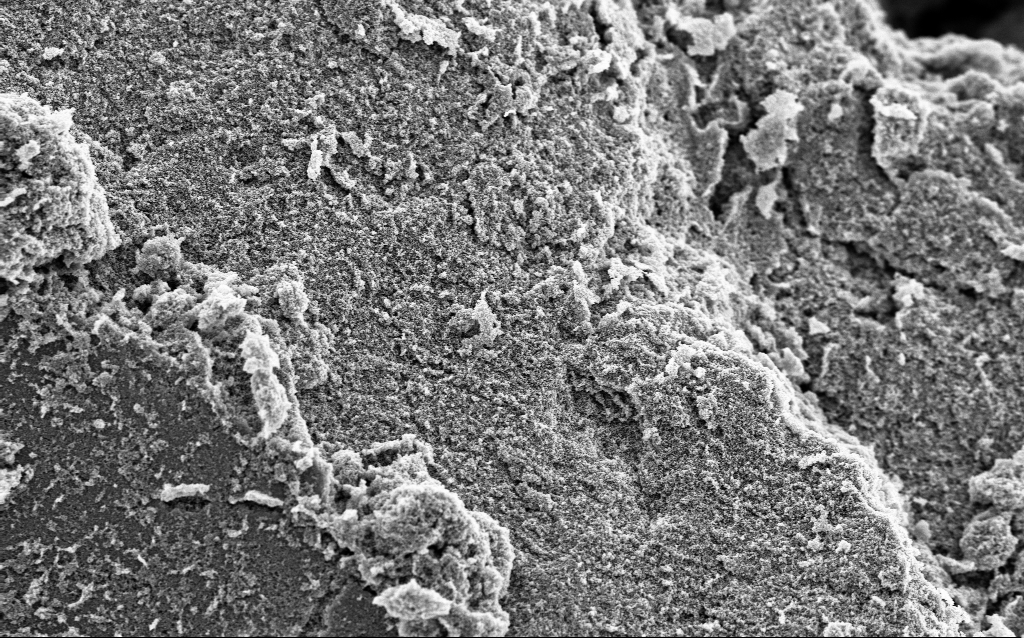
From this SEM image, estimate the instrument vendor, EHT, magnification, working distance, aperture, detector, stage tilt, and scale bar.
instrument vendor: Zeiss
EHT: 5 kV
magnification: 6.42 K X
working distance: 4.4 mm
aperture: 30 µm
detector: InLens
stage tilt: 0°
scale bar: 10000 nm